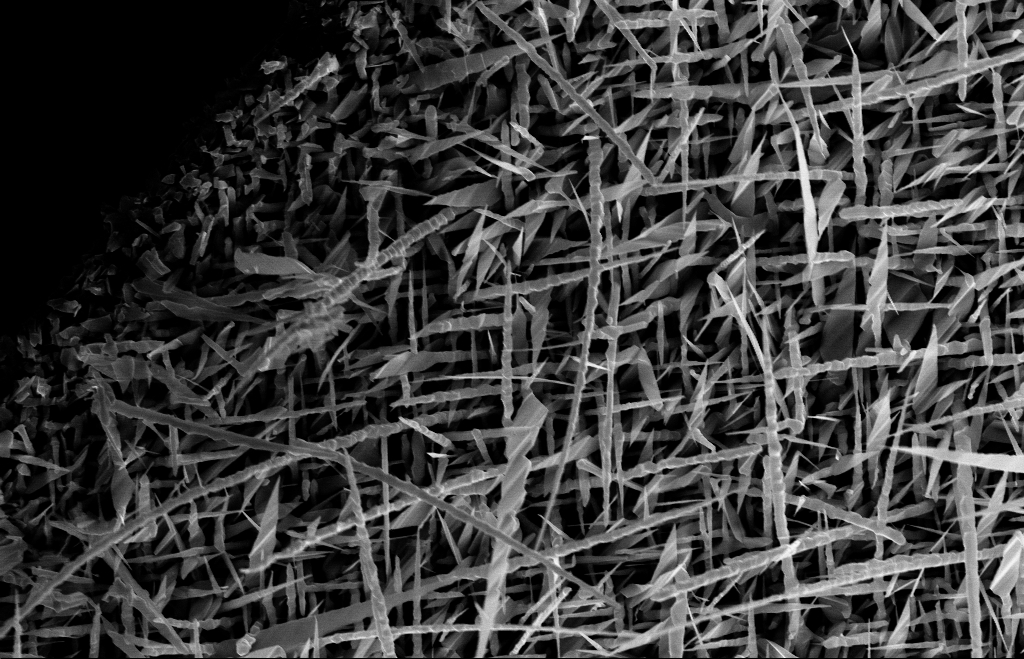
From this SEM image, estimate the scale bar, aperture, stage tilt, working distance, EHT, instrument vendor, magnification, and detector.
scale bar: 1000 nm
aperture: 30 µm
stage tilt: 0°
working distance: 9 mm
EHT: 10 kV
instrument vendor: Zeiss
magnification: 20 K X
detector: InLens